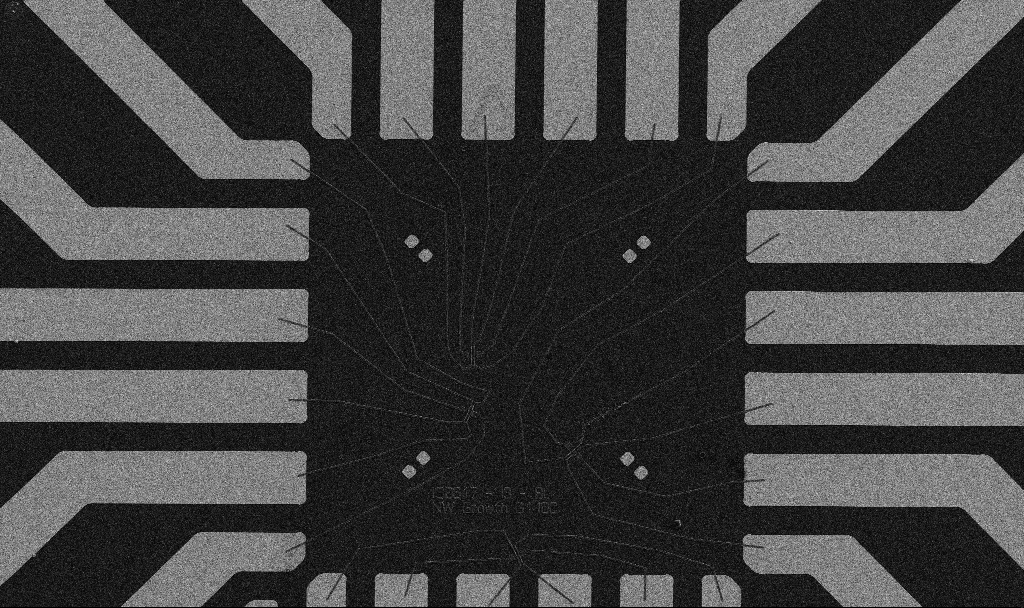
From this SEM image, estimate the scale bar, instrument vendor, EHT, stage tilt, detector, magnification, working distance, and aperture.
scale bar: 20000 nm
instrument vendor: Zeiss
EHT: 5 kV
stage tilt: -0°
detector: SE2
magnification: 1 K X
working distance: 10.7 mm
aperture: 30 µm